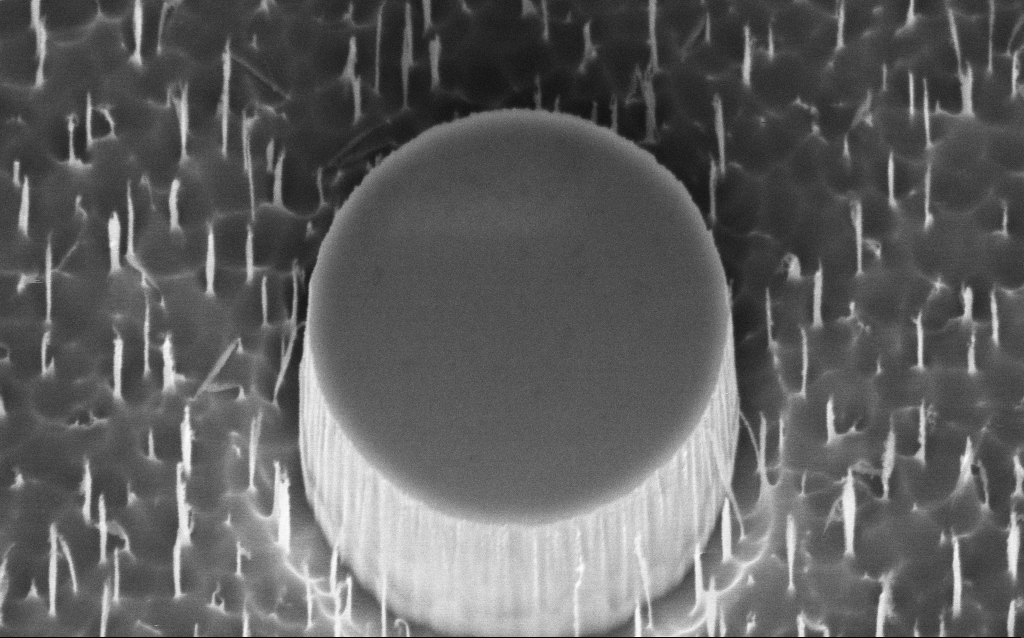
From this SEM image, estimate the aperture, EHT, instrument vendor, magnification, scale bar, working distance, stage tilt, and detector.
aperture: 30 µm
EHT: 3 kV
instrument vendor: Zeiss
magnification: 78.58 K X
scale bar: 200 nm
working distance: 8 mm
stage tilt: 45°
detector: InLens